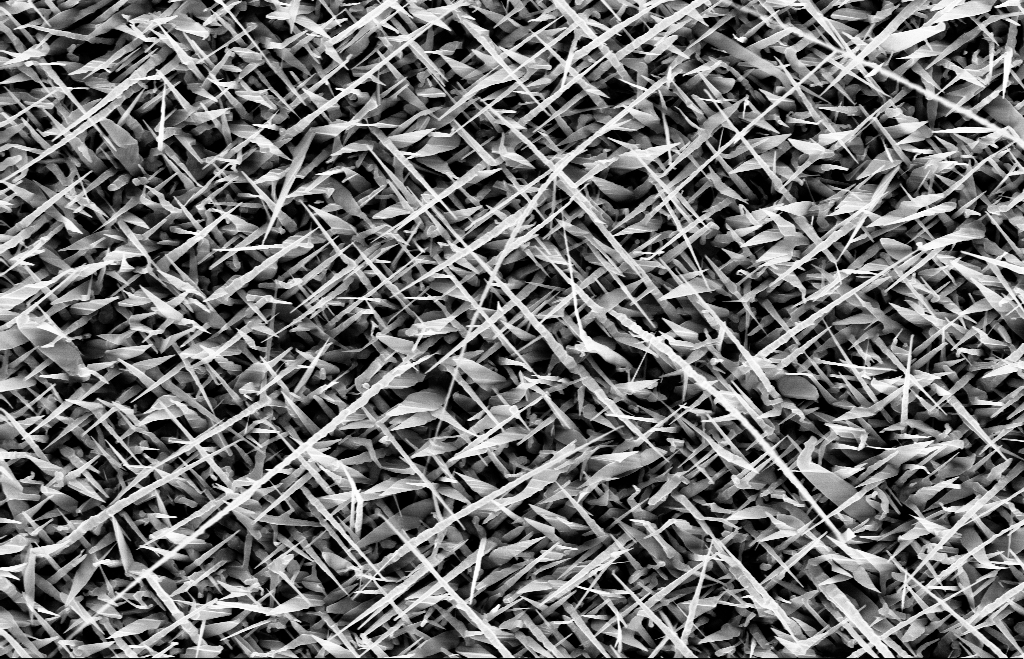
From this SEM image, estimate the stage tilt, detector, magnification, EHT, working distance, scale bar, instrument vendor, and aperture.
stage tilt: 0°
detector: InLens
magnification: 20 K X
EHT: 10 kV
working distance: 8 mm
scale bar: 1000 nm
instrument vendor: Zeiss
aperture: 30 µm